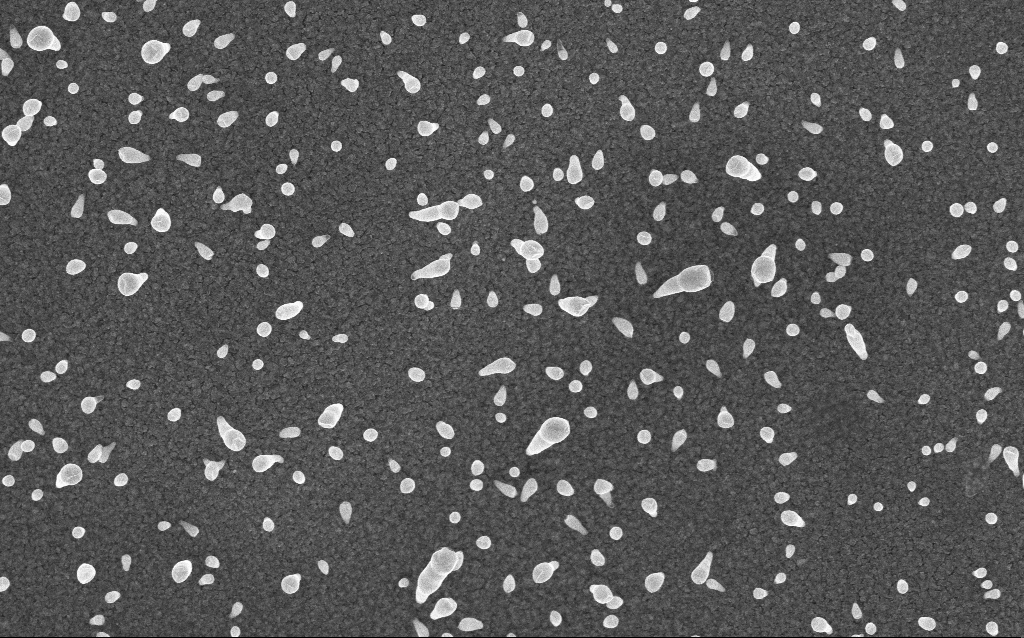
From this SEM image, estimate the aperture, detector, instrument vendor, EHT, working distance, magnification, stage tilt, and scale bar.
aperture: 30 µm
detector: InLens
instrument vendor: Zeiss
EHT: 5 kV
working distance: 4 mm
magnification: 53.96 K X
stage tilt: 0°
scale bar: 1000 nm